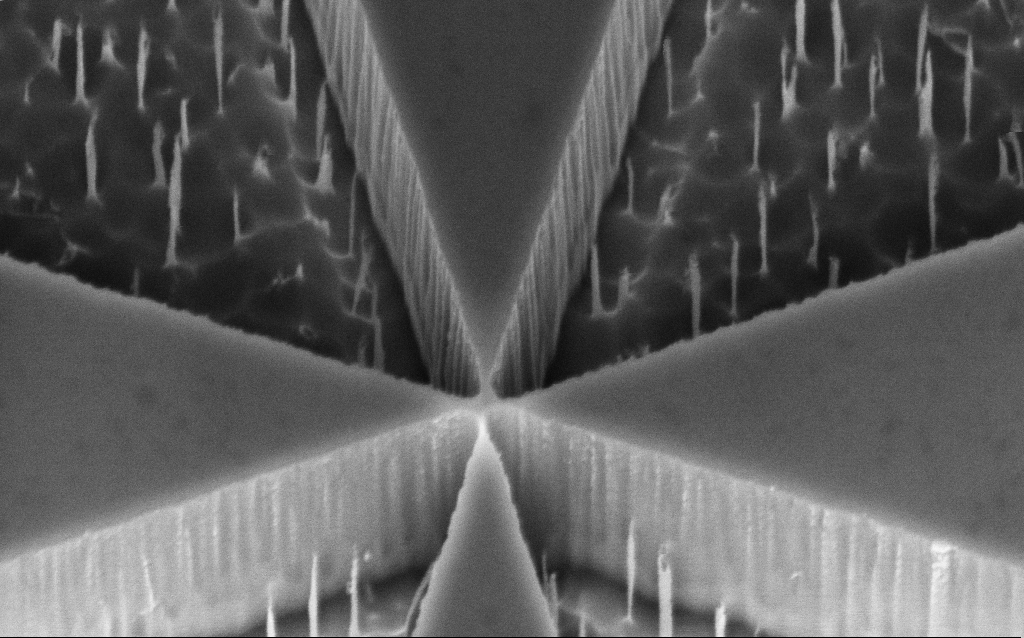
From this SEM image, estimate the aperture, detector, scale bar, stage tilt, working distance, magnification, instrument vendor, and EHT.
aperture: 30 µm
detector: InLens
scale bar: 200 nm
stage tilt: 45°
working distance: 8 mm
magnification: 90.89 K X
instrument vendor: Zeiss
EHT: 3 kV